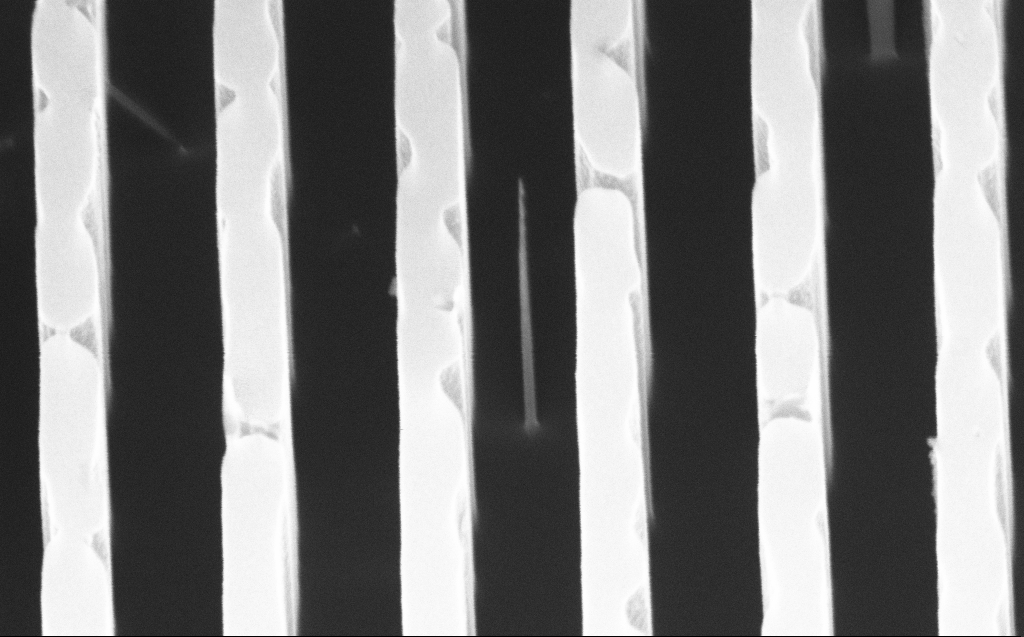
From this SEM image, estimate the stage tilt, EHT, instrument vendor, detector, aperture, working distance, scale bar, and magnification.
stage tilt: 45°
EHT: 10 kV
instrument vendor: Zeiss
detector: InLens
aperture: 30 µm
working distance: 7 mm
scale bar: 200 nm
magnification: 132.97 K X